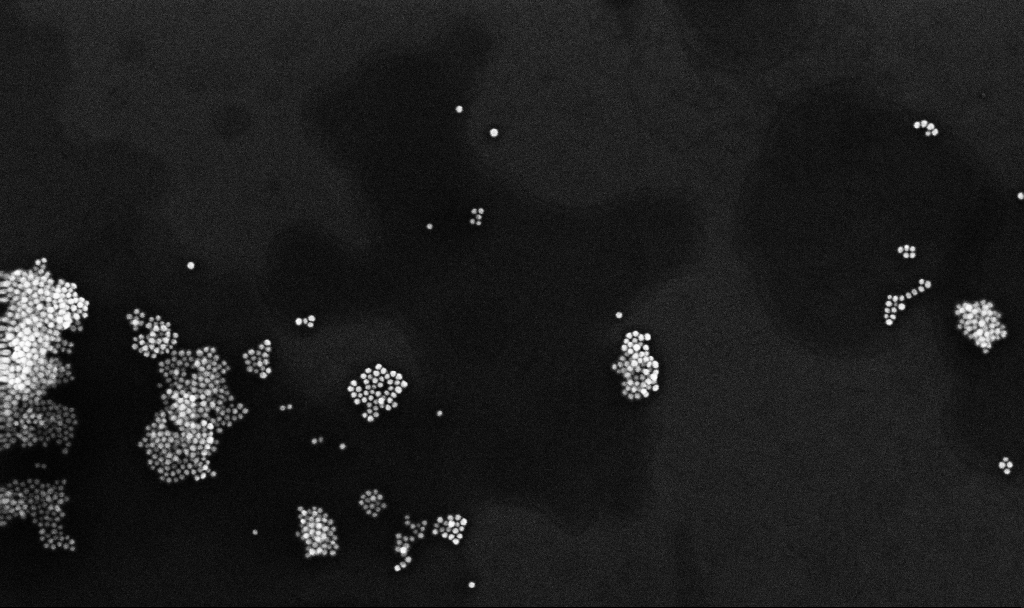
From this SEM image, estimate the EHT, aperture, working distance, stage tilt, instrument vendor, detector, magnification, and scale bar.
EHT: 10 kV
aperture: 30 µm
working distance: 3.3 mm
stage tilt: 0°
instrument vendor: Zeiss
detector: InLens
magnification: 110.72 K X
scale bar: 200 nm